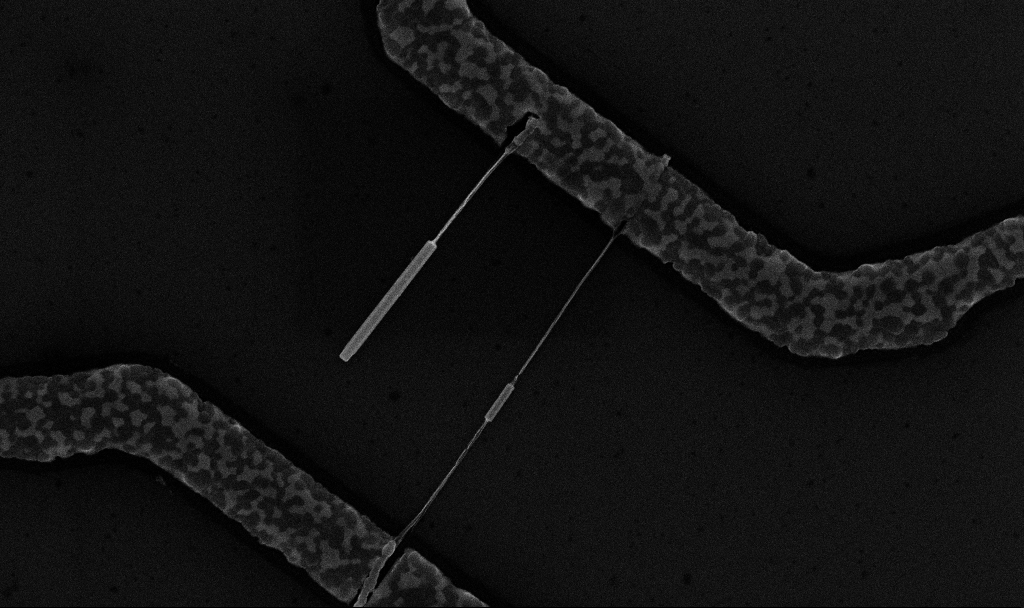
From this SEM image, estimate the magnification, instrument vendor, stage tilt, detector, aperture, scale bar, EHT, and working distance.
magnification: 35.31 K X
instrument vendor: Zeiss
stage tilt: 0°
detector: InLens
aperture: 30 µm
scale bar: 1000 nm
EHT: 10 kV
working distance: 6.7 mm